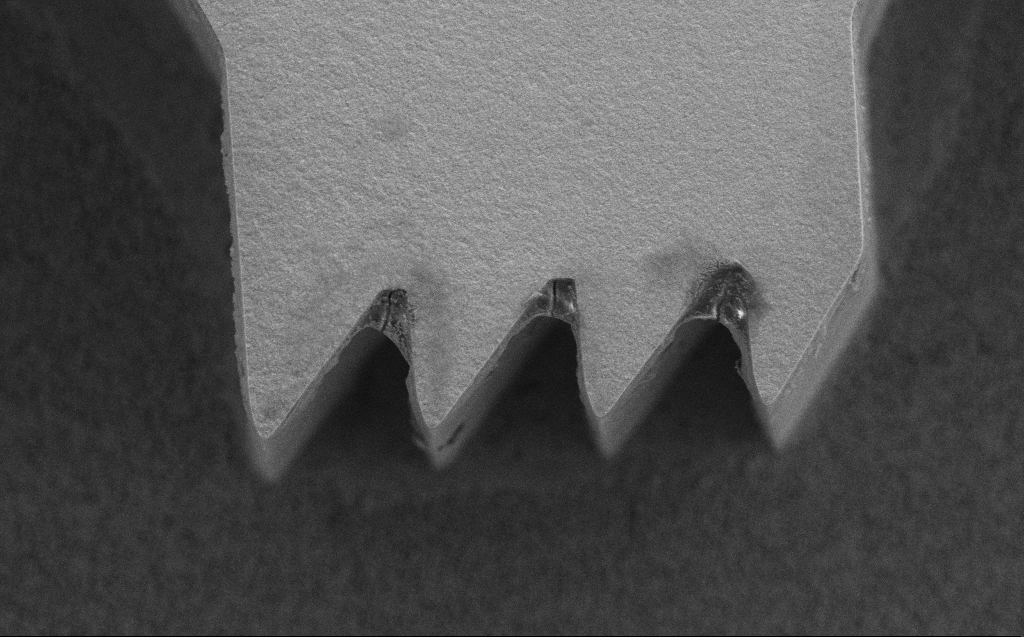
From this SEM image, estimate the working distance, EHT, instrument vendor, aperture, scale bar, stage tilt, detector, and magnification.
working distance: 7 mm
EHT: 5 kV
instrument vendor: Zeiss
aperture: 30 µm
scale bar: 10000 nm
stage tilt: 0°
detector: SE2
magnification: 5.45 K X